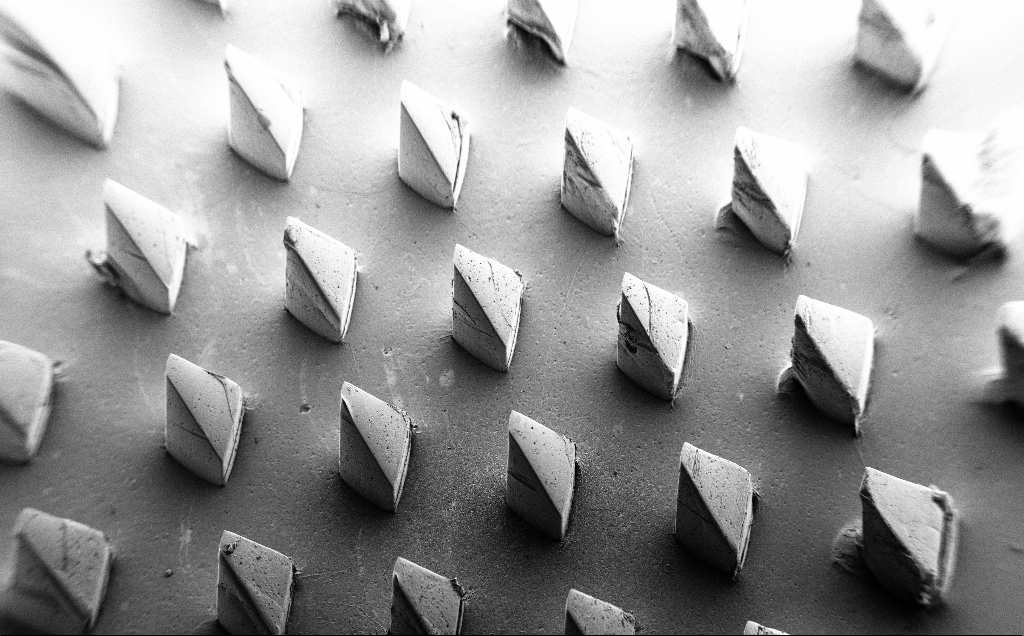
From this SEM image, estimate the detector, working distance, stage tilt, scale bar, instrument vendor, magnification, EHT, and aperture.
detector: SE2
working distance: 8 mm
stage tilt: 39°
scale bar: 1e+06 nm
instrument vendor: Zeiss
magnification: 0.067 K X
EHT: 5 kV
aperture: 30 µm